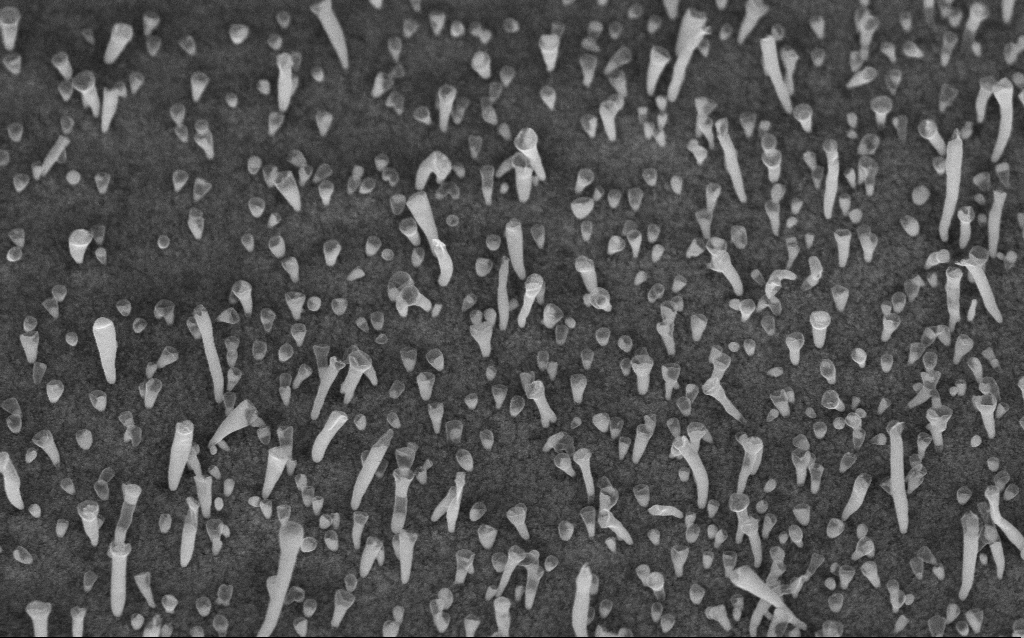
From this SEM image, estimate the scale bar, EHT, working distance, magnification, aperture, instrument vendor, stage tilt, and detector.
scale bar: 1000 nm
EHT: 5 kV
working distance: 6.7 mm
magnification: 50 K X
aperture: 30 µm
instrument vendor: Zeiss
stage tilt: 45°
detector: InLens